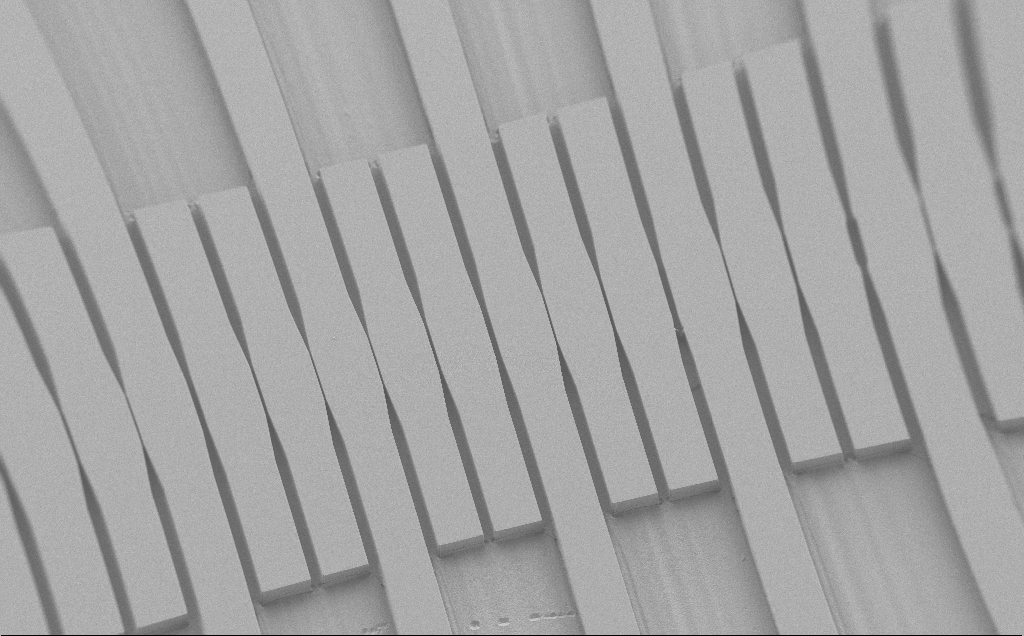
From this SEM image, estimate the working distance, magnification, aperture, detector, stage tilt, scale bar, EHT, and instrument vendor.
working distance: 6 mm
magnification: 0.206 K X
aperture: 30 µm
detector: SE2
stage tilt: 30°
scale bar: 100000 nm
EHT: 1.2 kV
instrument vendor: Zeiss